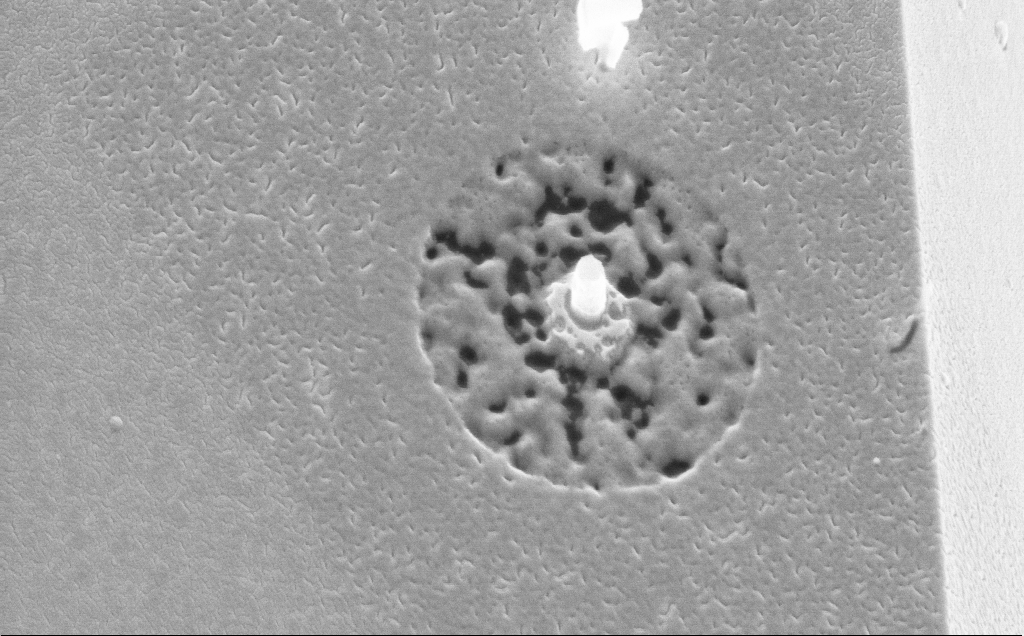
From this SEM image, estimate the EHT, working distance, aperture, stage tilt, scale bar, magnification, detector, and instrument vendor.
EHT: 10 kV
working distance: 3 mm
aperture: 30 µm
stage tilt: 44.2°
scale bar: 1000 nm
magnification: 41.93 K X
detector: InLens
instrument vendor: Zeiss